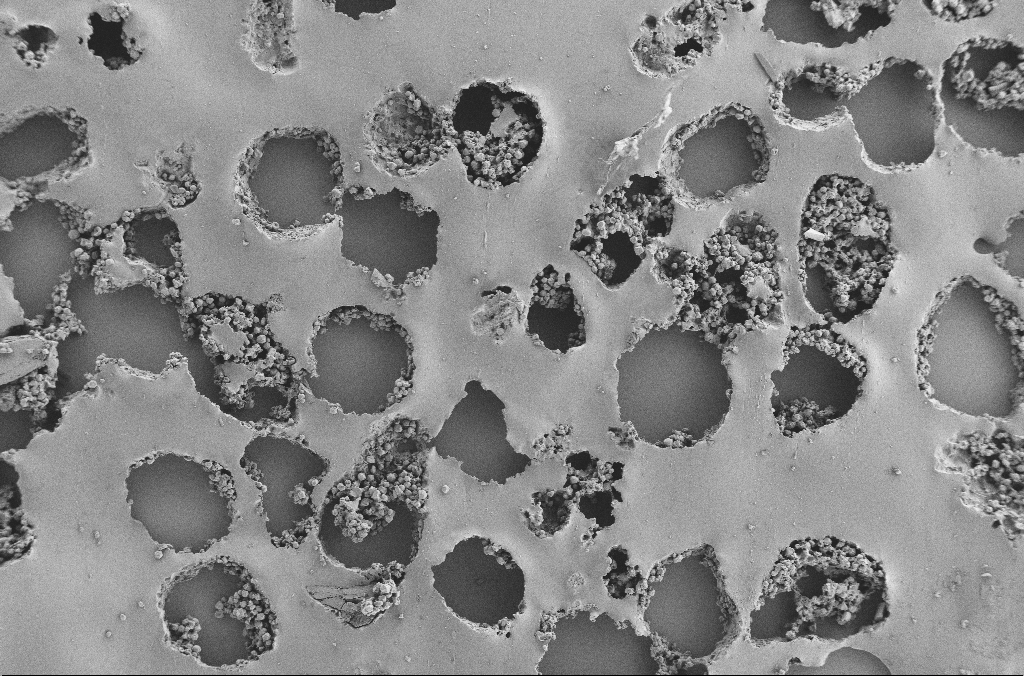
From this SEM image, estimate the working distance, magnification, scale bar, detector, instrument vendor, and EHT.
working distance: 3.7 mm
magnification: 0.2 K X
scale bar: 200000 nm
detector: SE2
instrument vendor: Zeiss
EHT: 2 kV